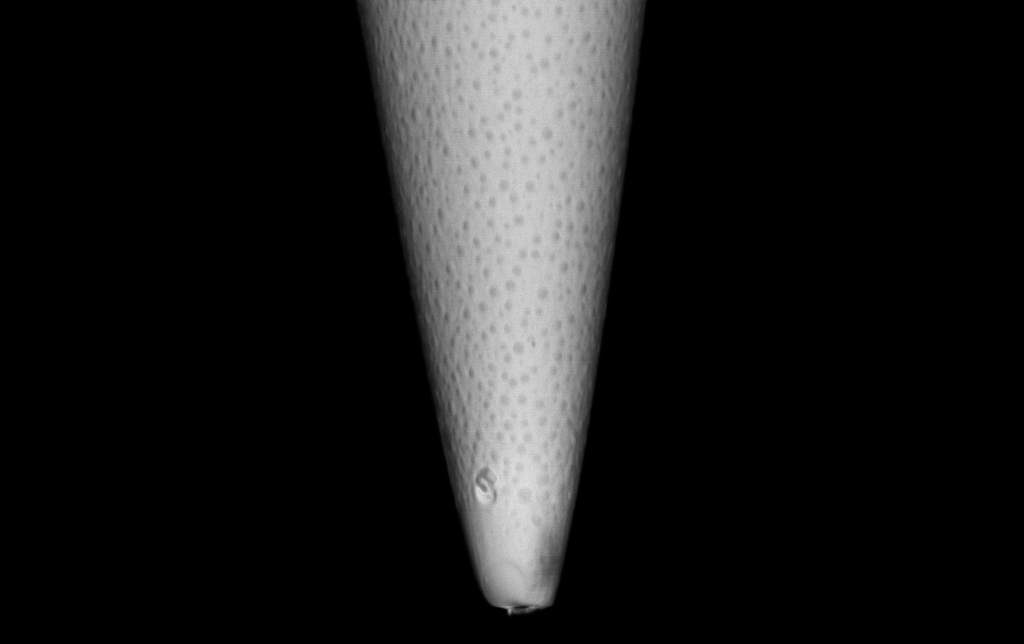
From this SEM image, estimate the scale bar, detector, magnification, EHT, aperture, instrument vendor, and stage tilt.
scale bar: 2000 nm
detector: InLens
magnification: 25 K X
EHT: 1 kV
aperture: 30 µm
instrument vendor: Zeiss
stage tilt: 0°